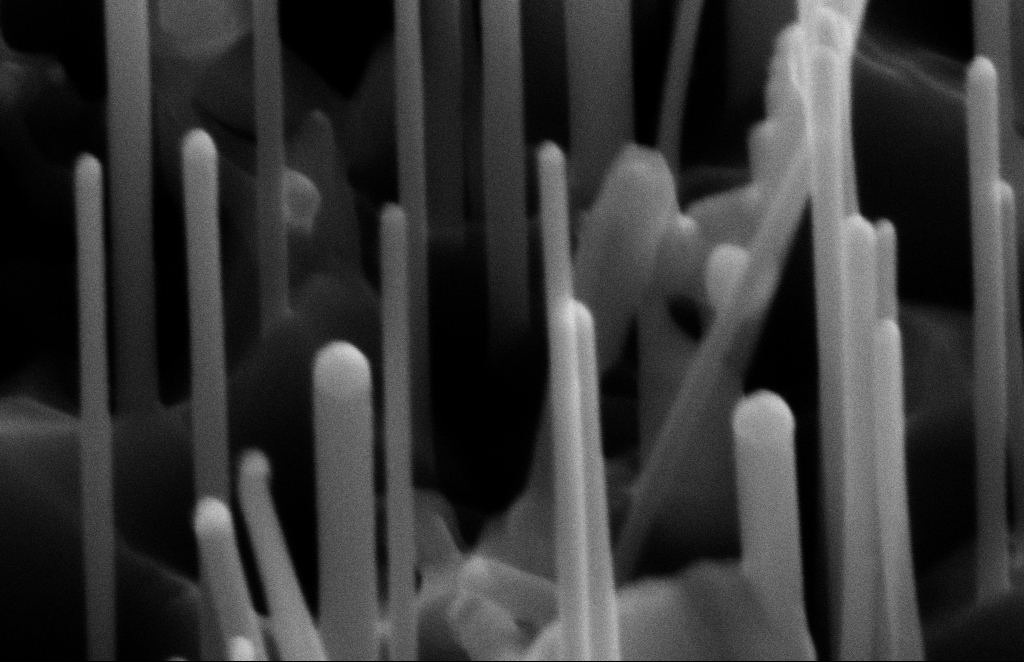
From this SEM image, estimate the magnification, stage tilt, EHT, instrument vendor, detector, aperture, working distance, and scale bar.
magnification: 300 K X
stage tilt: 45°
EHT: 10 kV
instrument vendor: Zeiss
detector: SE2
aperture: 20 µm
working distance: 15 mm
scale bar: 100 nm